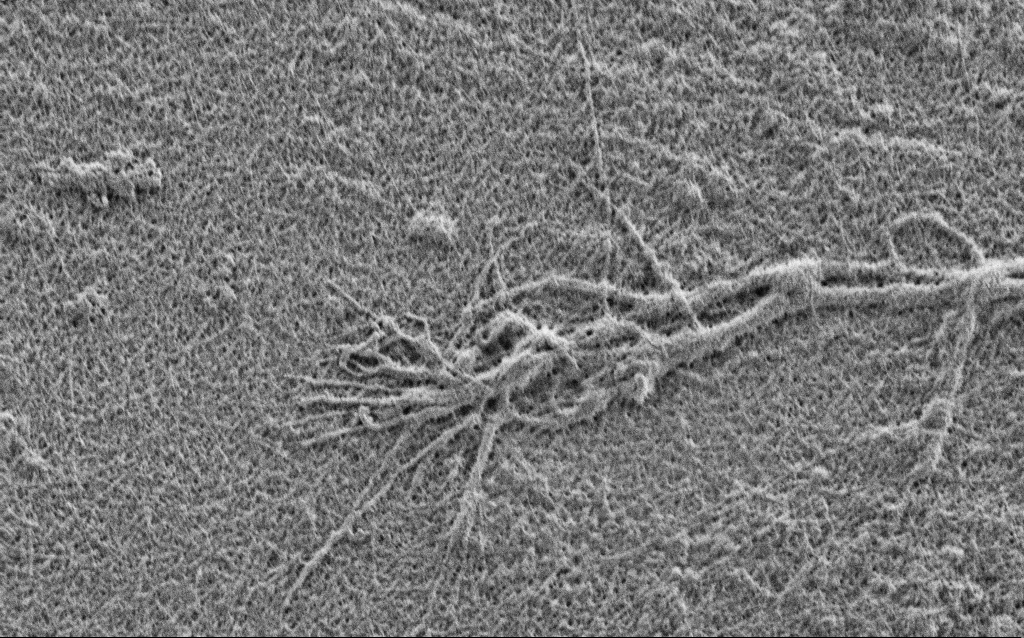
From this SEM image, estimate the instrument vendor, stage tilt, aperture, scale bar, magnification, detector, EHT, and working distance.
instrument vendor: Zeiss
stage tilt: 0°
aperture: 30 µm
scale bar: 2000 nm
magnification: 15 K X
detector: SE2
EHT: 1 kV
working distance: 4 mm